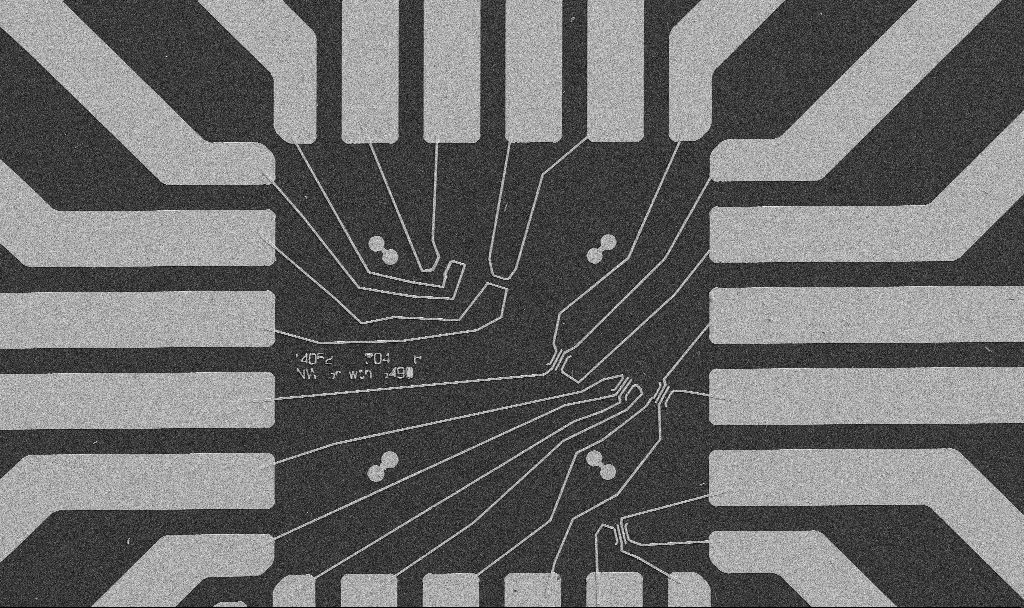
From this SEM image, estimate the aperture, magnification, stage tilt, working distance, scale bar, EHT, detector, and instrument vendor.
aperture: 30 µm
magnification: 1 K X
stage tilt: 0°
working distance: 10.7 mm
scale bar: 20000 nm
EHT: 5 kV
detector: SE2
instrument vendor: Zeiss